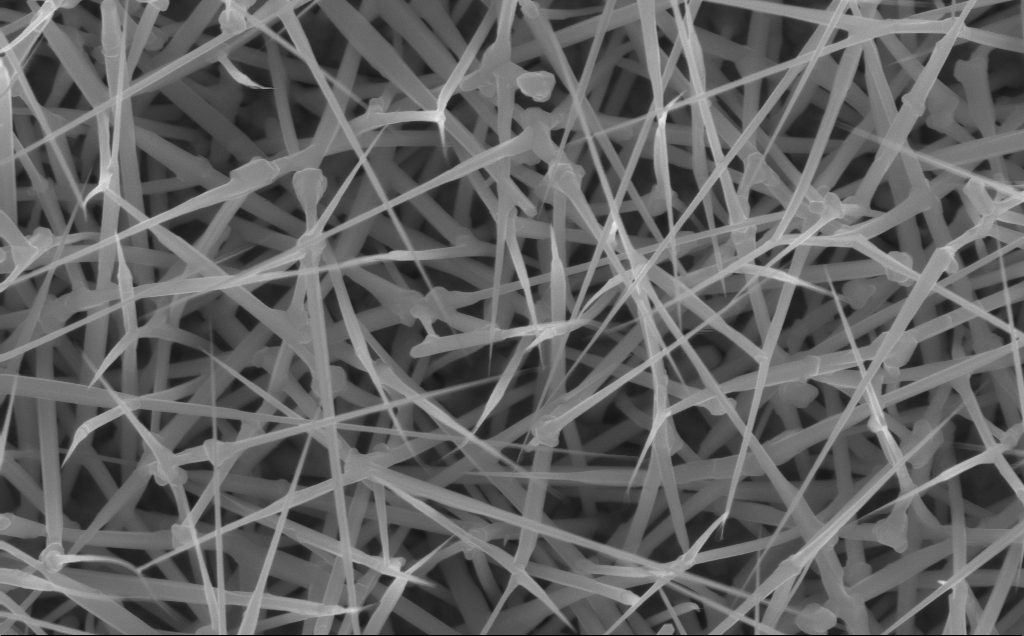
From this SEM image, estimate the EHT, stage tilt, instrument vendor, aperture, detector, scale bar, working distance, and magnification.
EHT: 10 kV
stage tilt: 0°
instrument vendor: Zeiss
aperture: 30 µm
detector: InLens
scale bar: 1000 nm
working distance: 6 mm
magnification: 40 K X